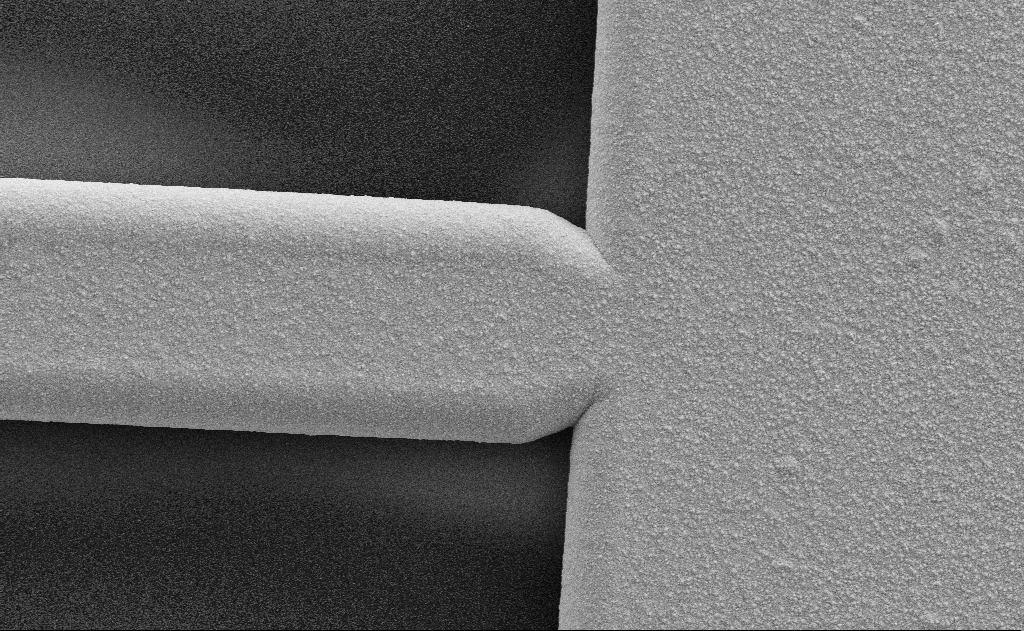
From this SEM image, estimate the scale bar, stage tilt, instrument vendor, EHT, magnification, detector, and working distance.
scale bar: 10000 nm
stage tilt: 0°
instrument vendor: Zeiss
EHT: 10 kV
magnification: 4.96 K X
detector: SE2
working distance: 6 mm